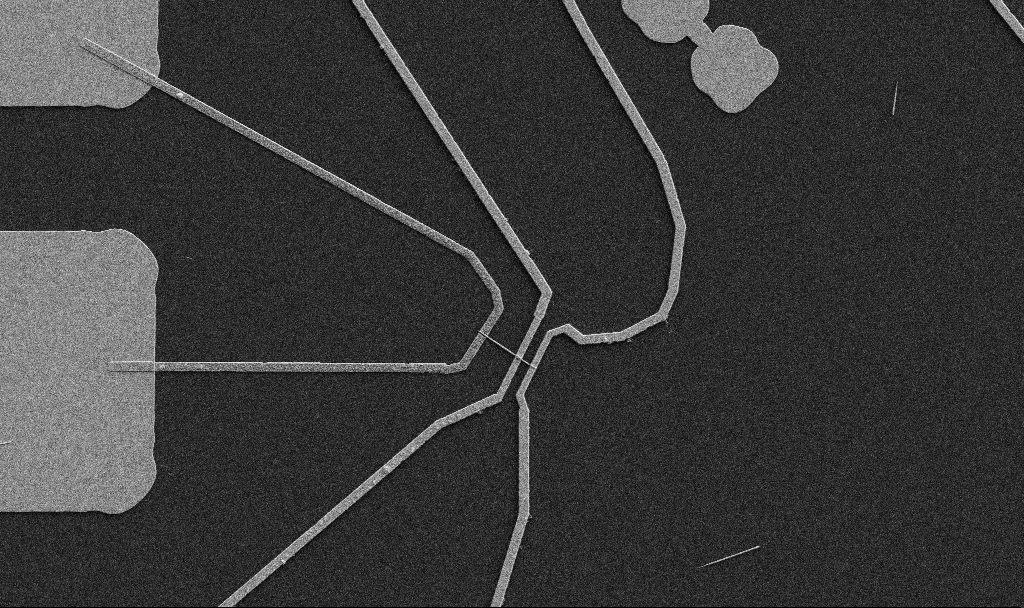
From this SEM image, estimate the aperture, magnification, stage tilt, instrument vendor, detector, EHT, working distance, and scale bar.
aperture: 30 µm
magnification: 5 K X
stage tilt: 0°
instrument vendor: Zeiss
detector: SE2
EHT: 5 kV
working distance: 10.7 mm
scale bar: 10000 nm